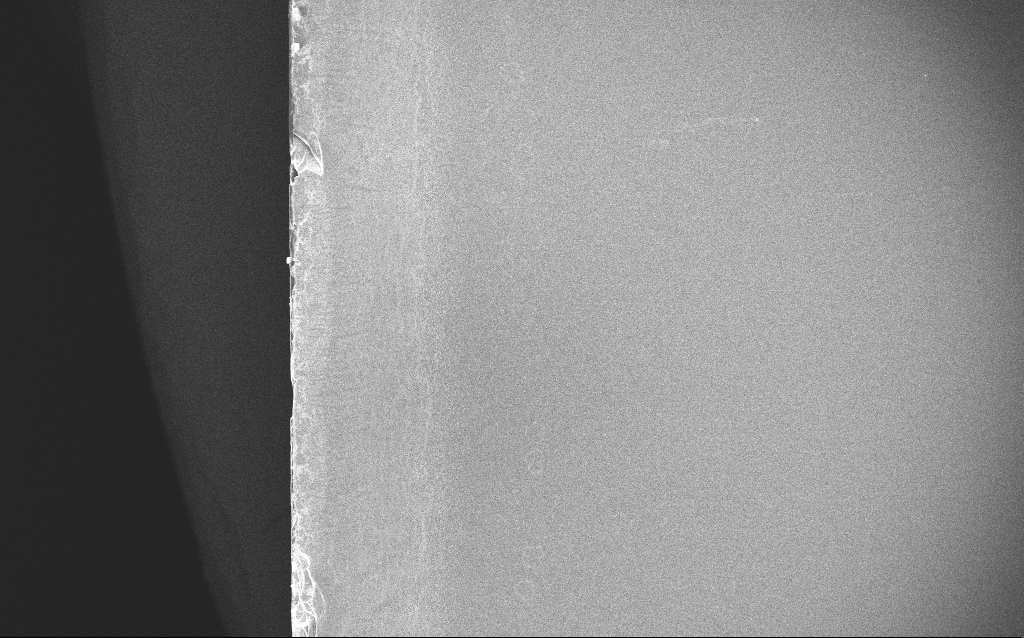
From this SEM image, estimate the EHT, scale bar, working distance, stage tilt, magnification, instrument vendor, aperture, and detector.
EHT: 20 kV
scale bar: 100000 nm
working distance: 1.7 mm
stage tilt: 0°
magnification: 0.2 K X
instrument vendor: Zeiss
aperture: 30 µm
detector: InLens